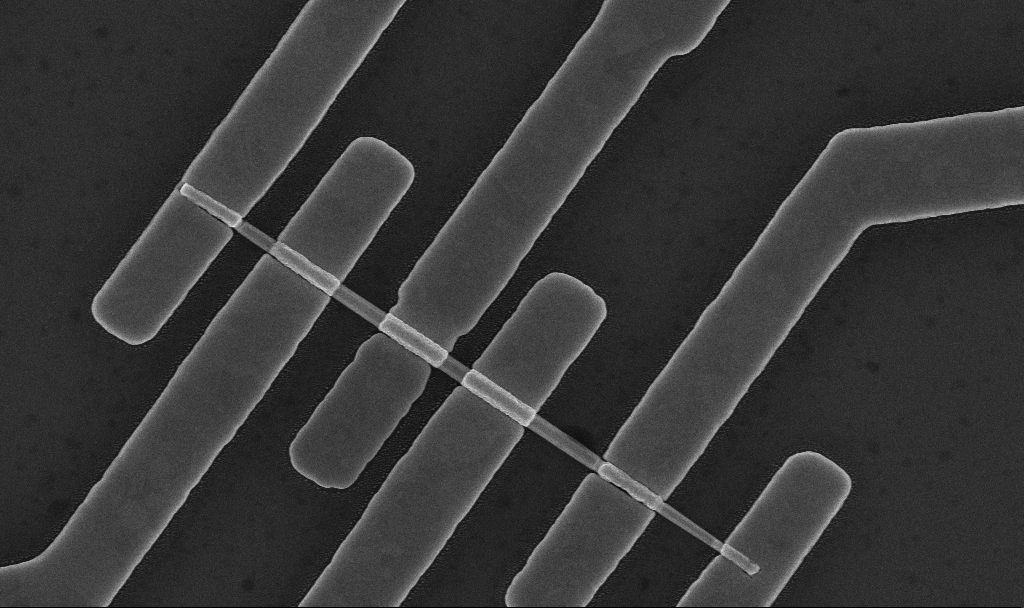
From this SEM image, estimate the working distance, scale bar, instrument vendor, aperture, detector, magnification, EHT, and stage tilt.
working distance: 7.6 mm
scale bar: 1000 nm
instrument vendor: Zeiss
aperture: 30 µm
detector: InLens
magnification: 41.93 K X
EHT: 10 kV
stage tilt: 0°